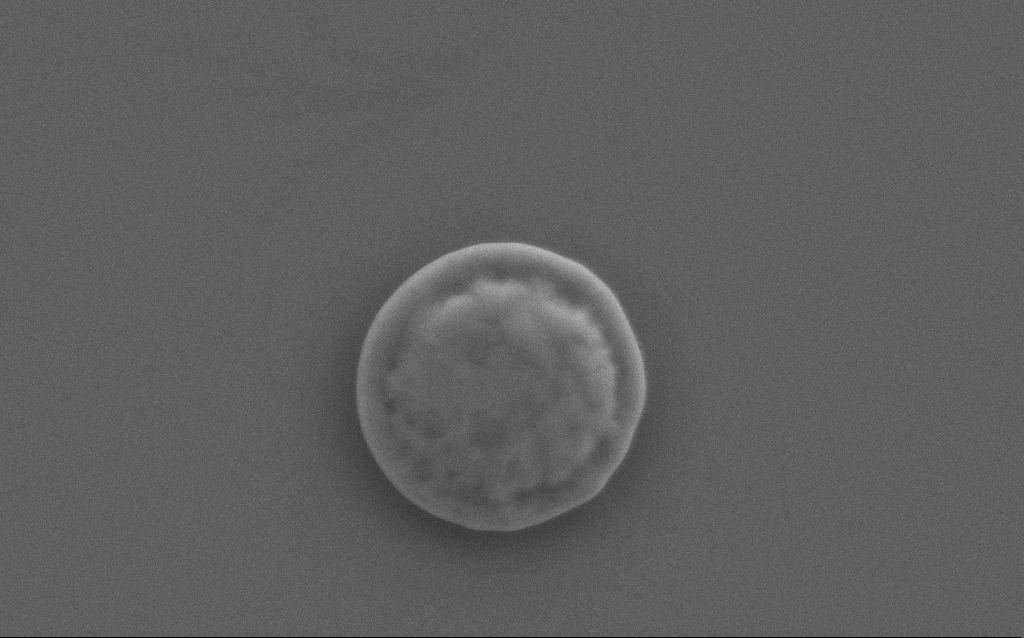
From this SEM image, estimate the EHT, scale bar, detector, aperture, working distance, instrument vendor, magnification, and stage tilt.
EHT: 5 kV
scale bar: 1000 nm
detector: SE2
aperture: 30 µm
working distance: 4 mm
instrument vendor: Zeiss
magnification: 64.05 K X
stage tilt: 0°